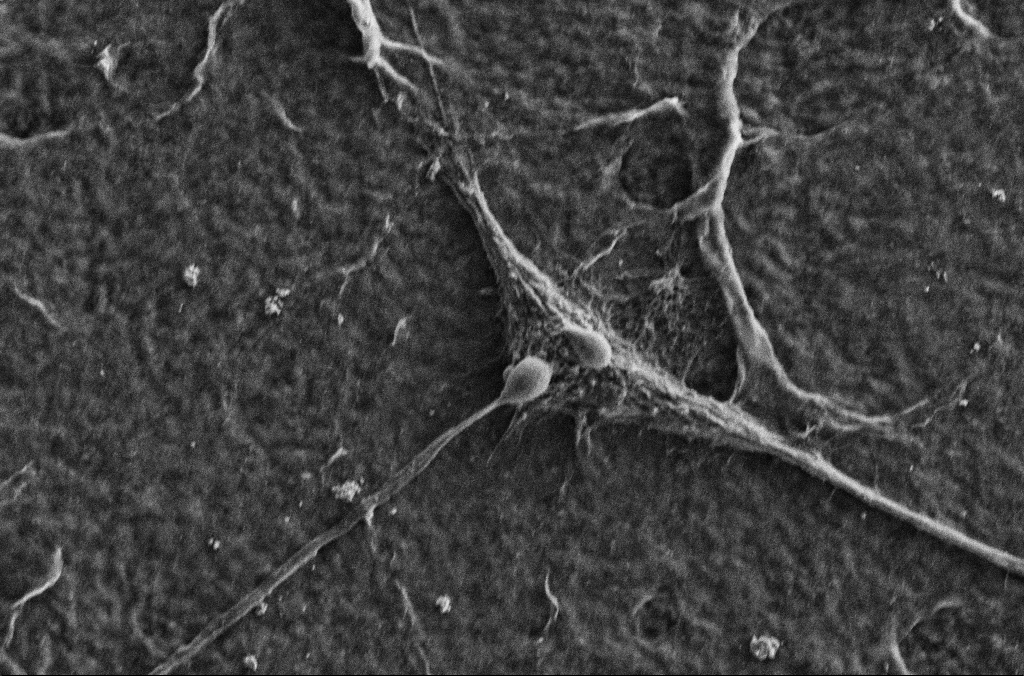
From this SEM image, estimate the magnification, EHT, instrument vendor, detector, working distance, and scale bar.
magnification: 2.5 K X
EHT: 5 kV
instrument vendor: Zeiss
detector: SE2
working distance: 2.9 mm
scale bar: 10000 nm